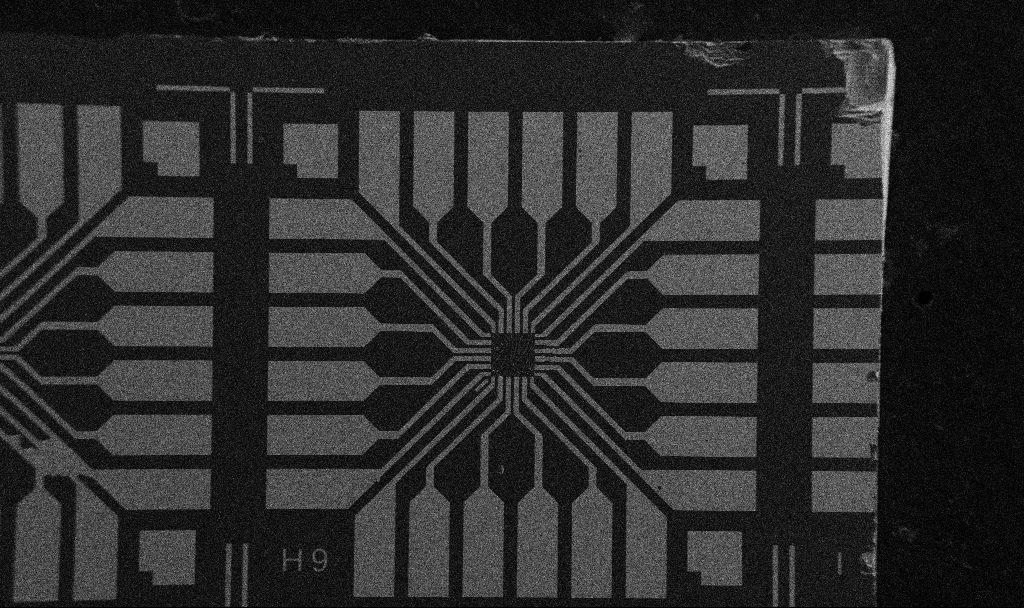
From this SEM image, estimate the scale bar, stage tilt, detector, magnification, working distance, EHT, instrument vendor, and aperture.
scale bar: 200000 nm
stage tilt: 0°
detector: SE2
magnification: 0.1 K X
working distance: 10.7 mm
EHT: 5 kV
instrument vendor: Zeiss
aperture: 30 µm